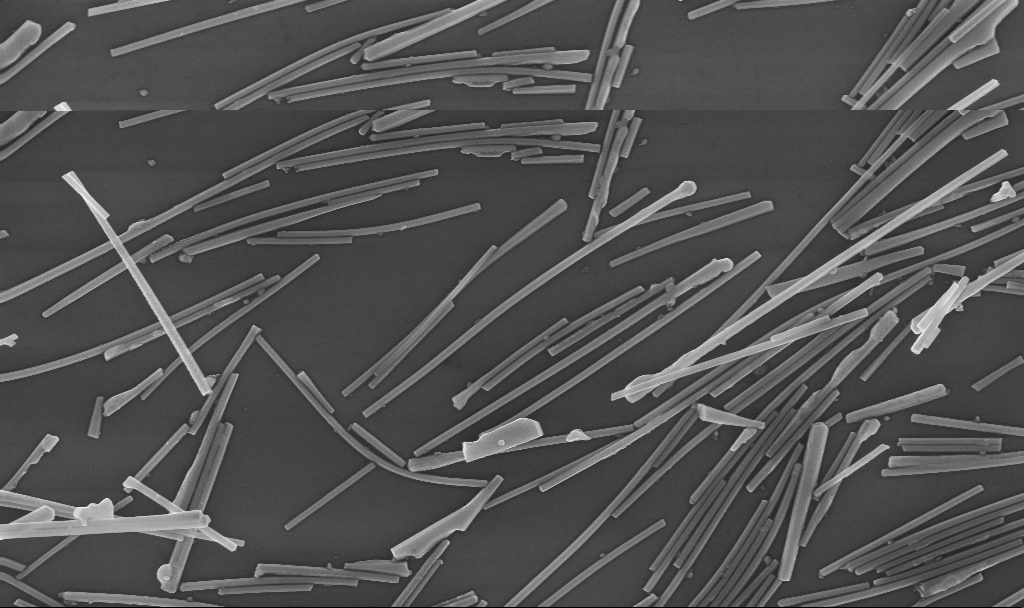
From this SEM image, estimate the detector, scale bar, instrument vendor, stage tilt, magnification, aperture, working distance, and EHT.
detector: InLens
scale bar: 2000 nm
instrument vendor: Zeiss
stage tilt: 0°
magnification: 26.17 K X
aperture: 30 µm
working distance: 6.7 mm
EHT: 10 kV